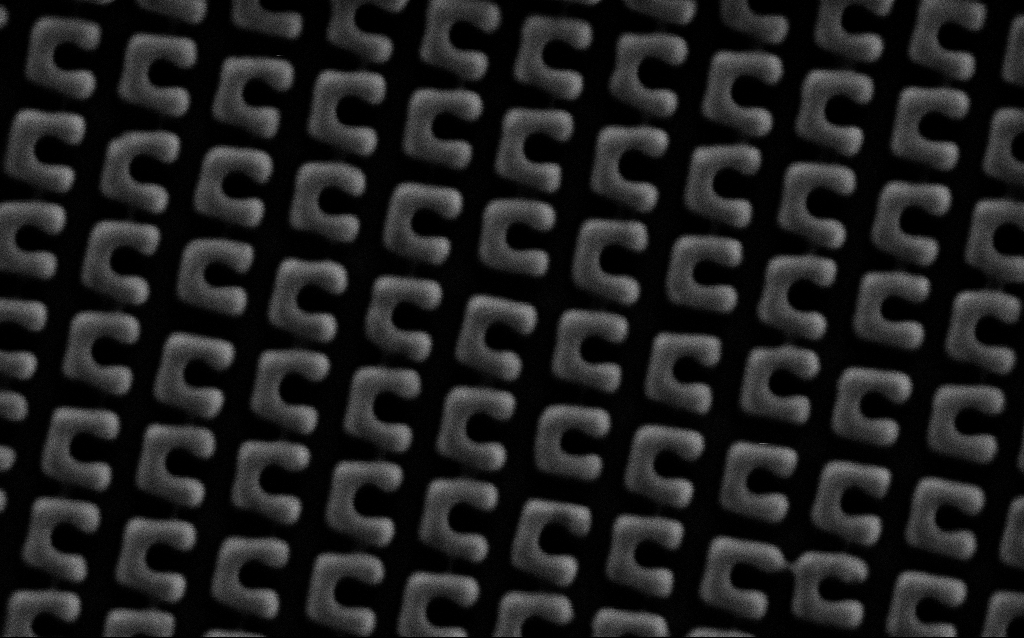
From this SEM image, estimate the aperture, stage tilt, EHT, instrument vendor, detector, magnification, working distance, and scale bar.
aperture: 30 µm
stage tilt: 0°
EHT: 1.5 kV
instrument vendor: Zeiss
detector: SE2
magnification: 75.6 K X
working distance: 7.7 mm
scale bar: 200 nm